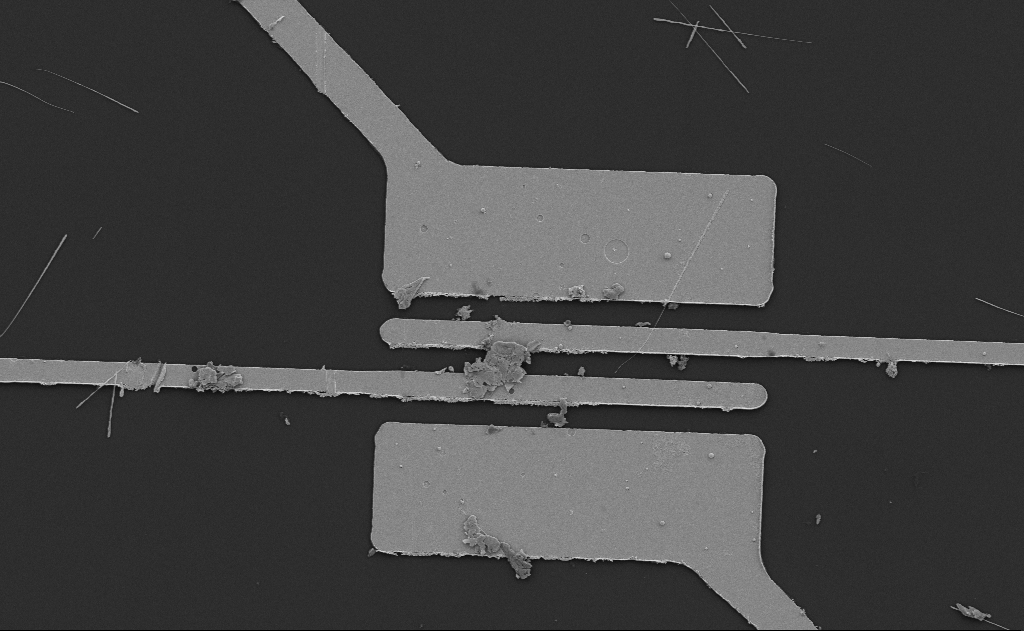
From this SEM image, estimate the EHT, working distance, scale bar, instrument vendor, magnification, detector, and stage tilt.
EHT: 5 kV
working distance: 13 mm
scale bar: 10000 nm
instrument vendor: Zeiss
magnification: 4.8 K X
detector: SE2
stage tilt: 0°